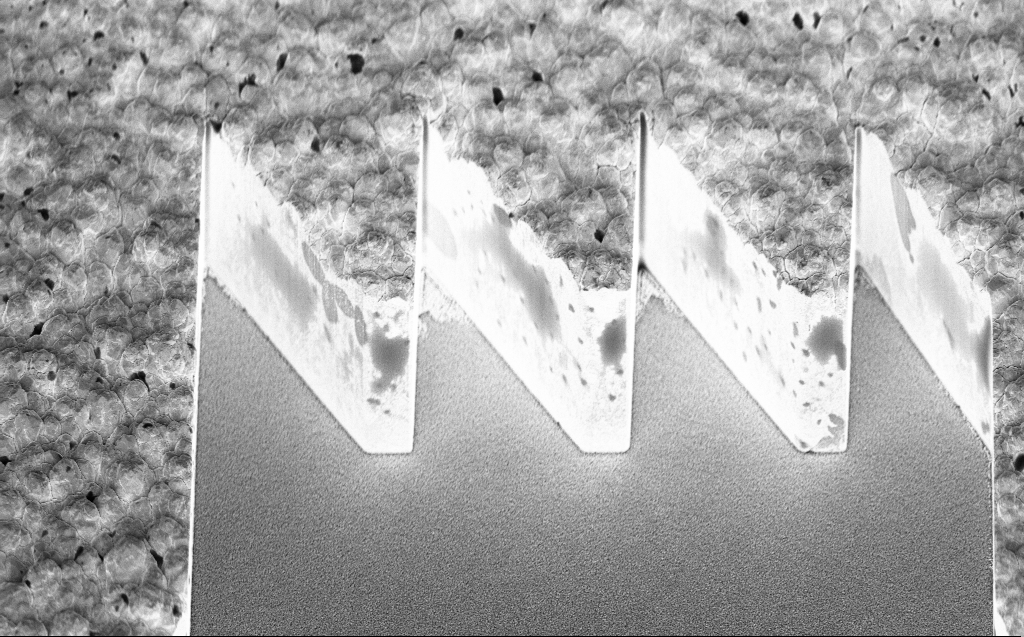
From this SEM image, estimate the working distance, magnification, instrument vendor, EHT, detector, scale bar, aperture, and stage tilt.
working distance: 6 mm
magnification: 7.05 K X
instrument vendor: Zeiss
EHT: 5 kV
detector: InLens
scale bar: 10000 nm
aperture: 30 µm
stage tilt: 45°